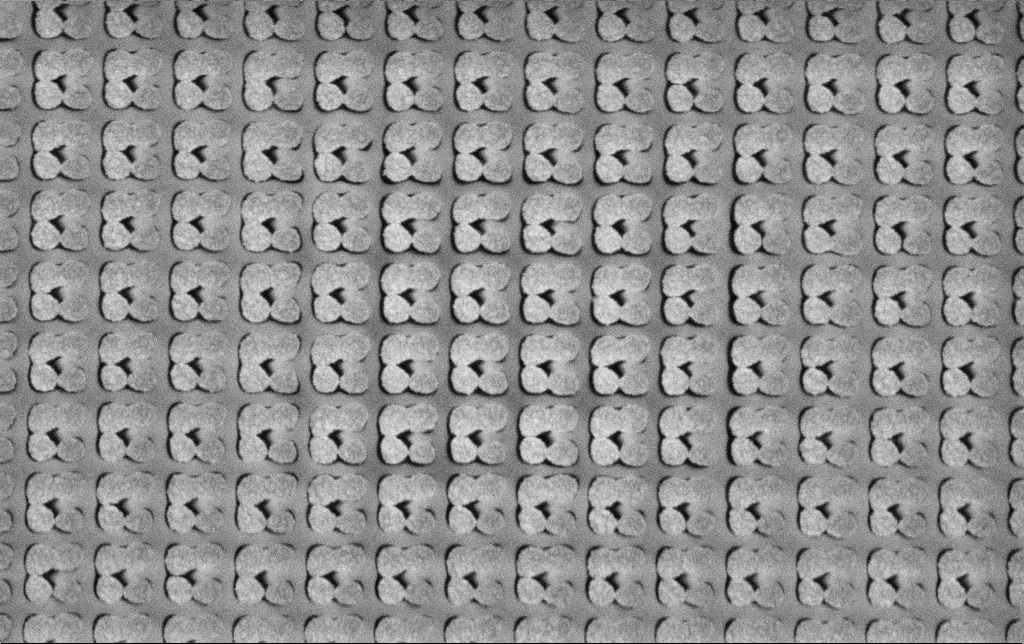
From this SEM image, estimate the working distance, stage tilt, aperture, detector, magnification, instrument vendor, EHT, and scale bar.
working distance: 6.2 mm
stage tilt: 0°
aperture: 30 µm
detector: SE2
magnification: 55.86 K X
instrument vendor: Zeiss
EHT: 3 kV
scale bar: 1000 nm